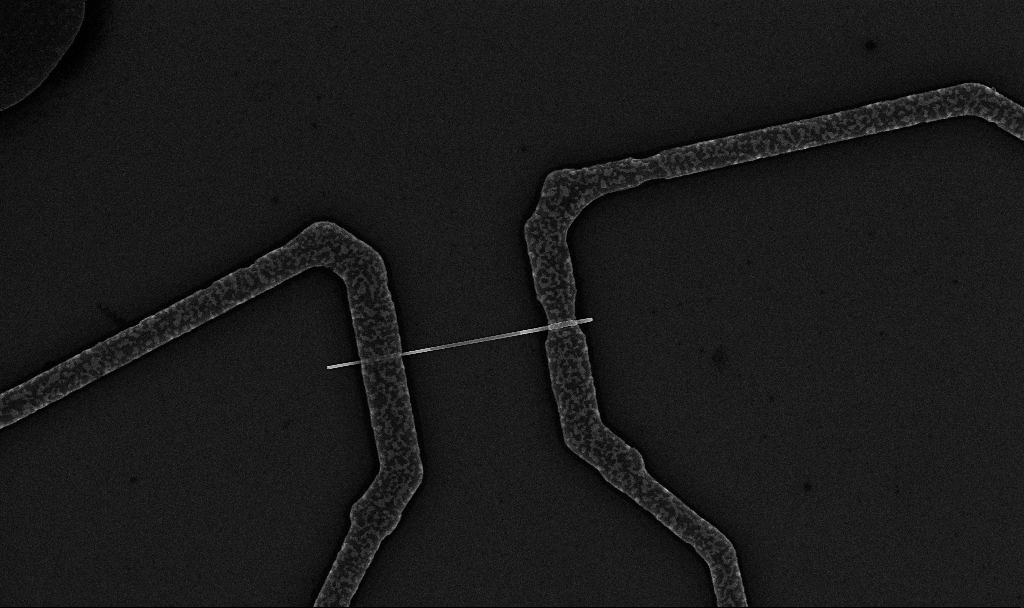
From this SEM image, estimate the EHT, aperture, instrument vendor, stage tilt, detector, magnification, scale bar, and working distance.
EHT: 10 kV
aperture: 30 µm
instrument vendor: Zeiss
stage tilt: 0°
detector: InLens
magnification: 15.63 K X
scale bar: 2000 nm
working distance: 6.7 mm